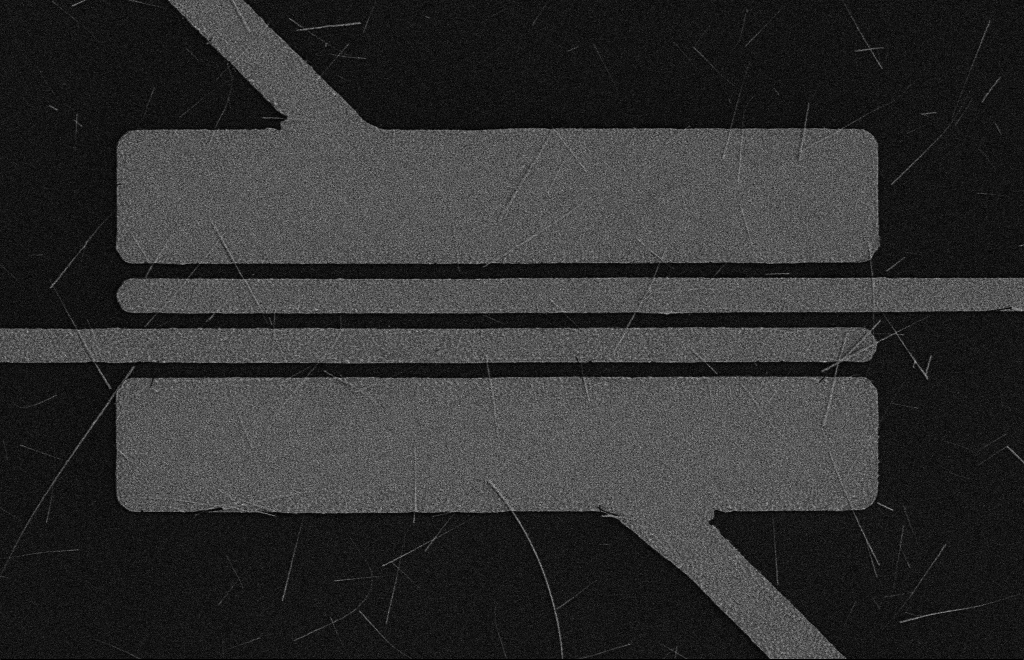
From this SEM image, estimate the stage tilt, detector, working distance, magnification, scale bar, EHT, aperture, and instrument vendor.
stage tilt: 0°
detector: SE2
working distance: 16 mm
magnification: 4.58 K X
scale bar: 10000 nm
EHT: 5 kV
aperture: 10 µm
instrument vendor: Zeiss